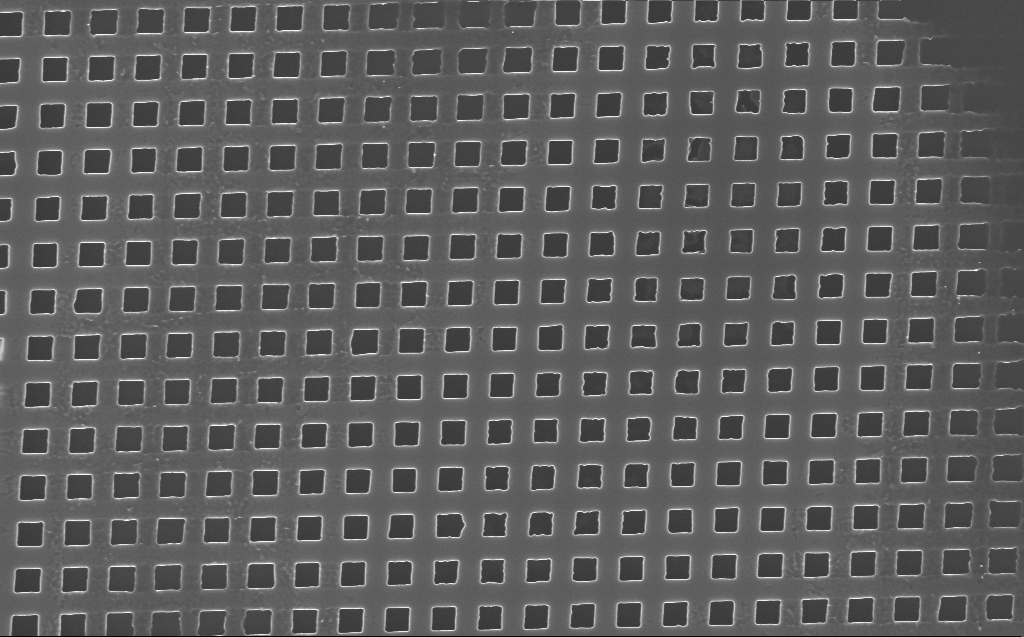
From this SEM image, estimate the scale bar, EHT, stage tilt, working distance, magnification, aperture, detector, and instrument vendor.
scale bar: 2000 nm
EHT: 10 kV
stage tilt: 0°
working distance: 4 mm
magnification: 34.52 K X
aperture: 30 µm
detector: InLens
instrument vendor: Zeiss